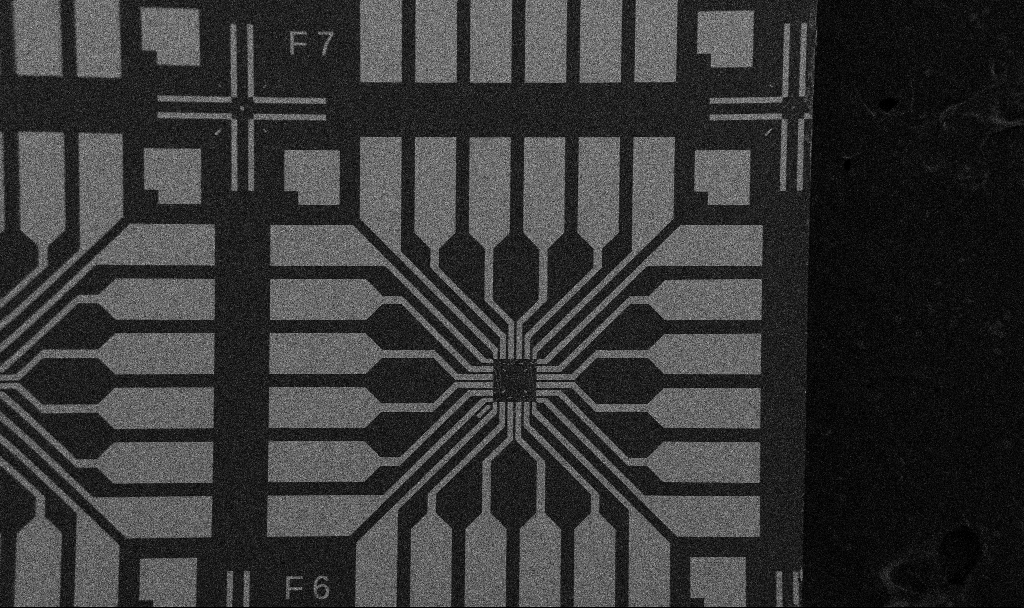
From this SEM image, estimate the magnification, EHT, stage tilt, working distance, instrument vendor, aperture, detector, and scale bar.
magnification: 0.1 K X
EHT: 5 kV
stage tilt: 0°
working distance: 10.7 mm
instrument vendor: Zeiss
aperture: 30 µm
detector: SE2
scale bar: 200000 nm